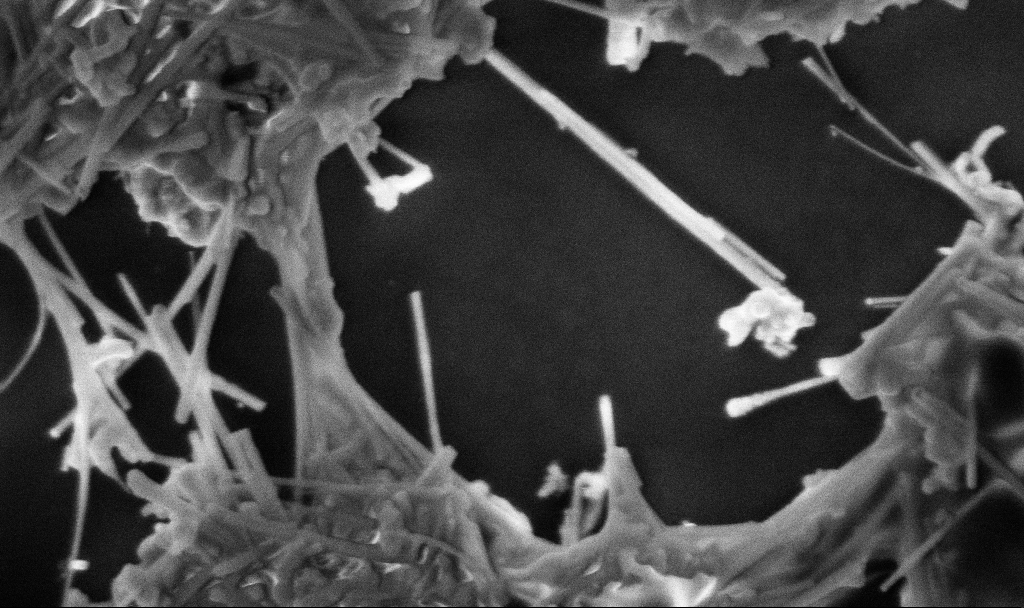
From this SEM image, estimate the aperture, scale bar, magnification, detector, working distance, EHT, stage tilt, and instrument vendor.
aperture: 30 µm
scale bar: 200 nm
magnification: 99.54 K X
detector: InLens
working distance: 3.3 mm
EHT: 3 kV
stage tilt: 0°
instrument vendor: Zeiss